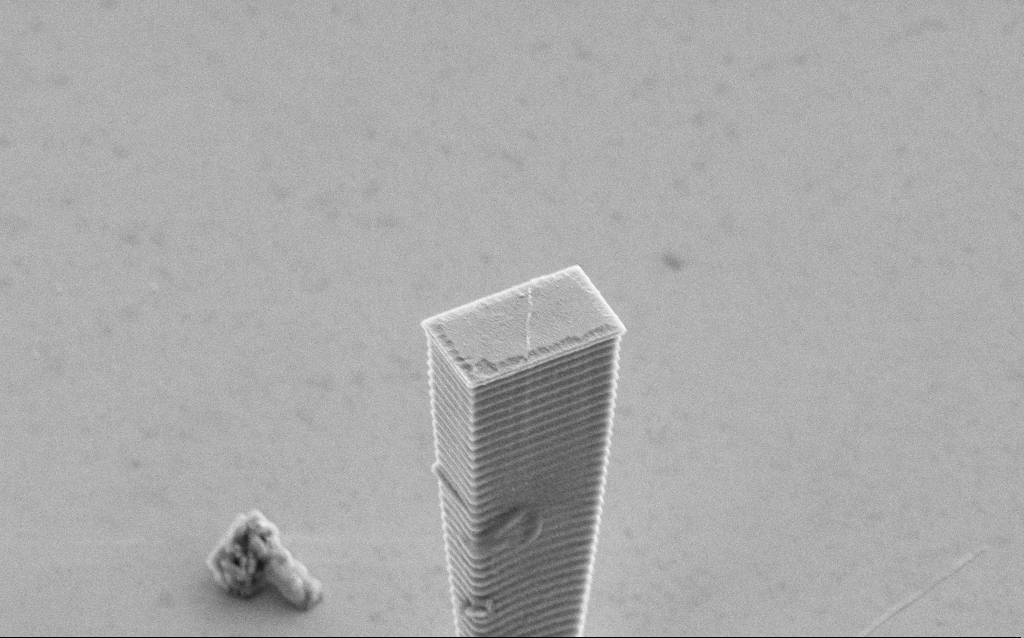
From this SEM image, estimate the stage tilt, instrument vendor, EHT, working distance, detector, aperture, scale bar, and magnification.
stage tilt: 45°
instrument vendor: Zeiss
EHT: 5 kV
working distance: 12 mm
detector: SE2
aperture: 30 µm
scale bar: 2000 nm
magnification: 13.31 K X